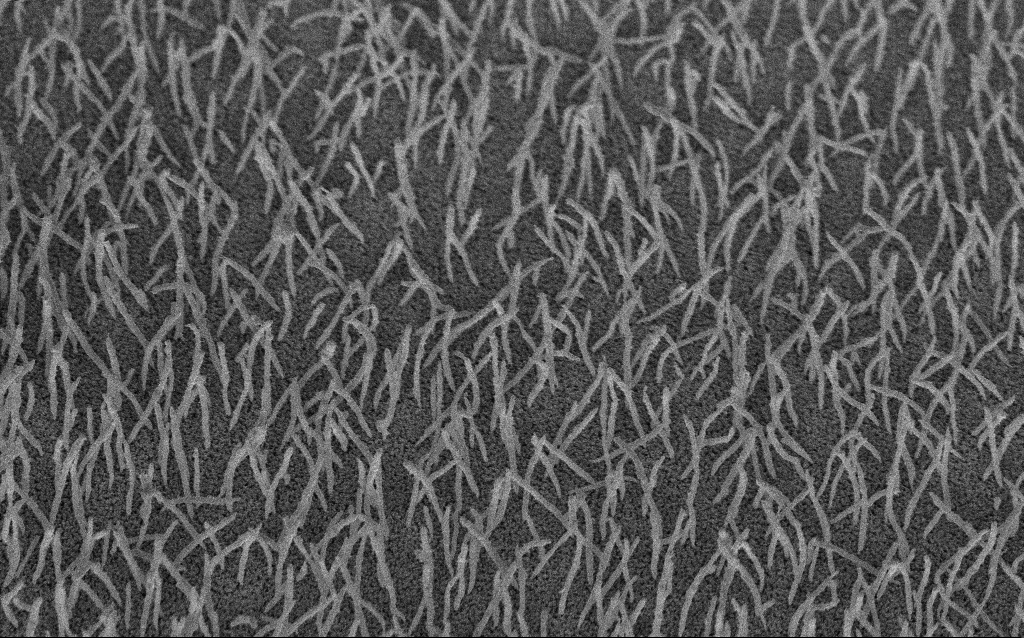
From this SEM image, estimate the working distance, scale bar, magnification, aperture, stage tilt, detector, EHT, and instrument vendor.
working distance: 8.2 mm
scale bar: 1000 nm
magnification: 20 K X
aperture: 30 µm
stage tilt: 45°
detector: InLens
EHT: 5 kV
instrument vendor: Zeiss